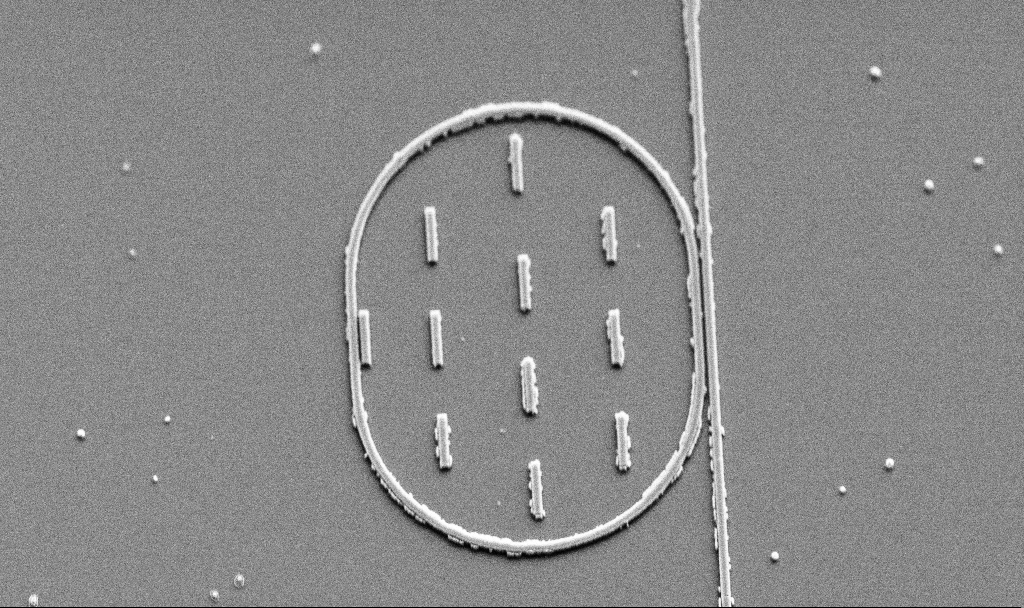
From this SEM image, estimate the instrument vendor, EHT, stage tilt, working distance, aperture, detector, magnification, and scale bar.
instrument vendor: Zeiss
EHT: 5 kV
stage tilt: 45°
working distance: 10 mm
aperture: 30 µm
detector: SE2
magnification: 6.47 K X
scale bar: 10000 nm